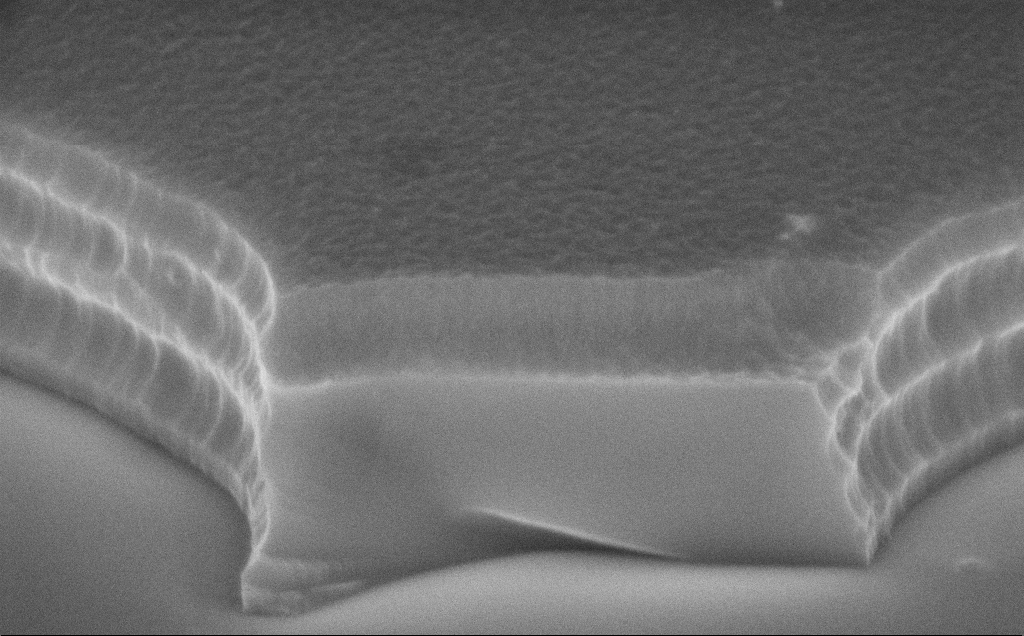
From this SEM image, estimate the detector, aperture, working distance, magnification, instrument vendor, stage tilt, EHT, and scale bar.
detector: InLens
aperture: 30 µm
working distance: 12 mm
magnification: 44.49 K X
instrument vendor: Zeiss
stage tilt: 70°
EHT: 8 kV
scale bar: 1000 nm